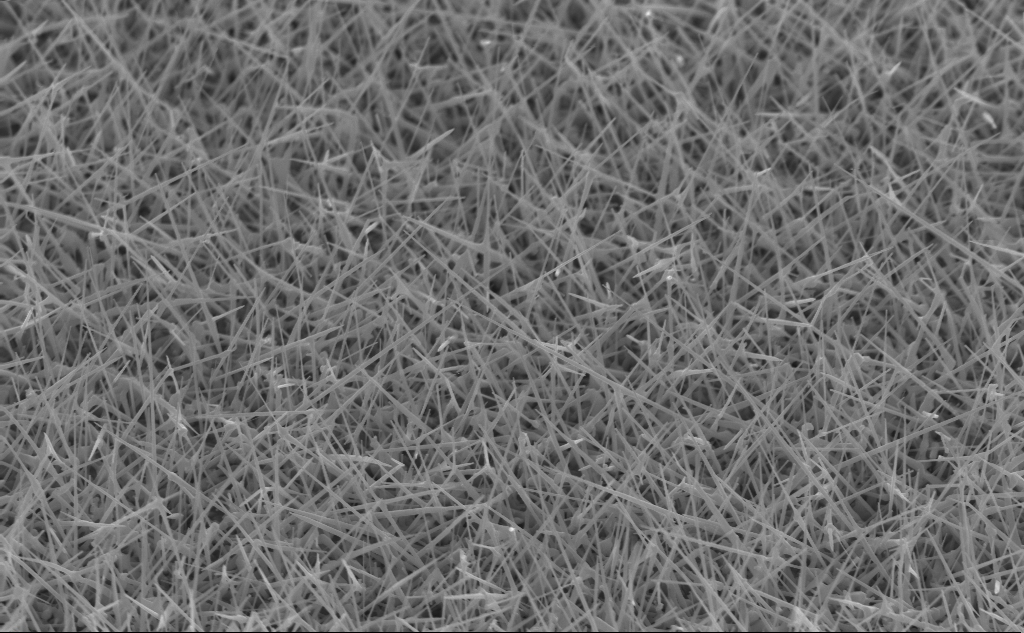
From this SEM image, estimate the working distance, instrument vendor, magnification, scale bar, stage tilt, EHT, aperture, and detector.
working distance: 4 mm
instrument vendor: Zeiss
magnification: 20 K X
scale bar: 2000 nm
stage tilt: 45°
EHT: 10 kV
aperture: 30 µm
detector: InLens